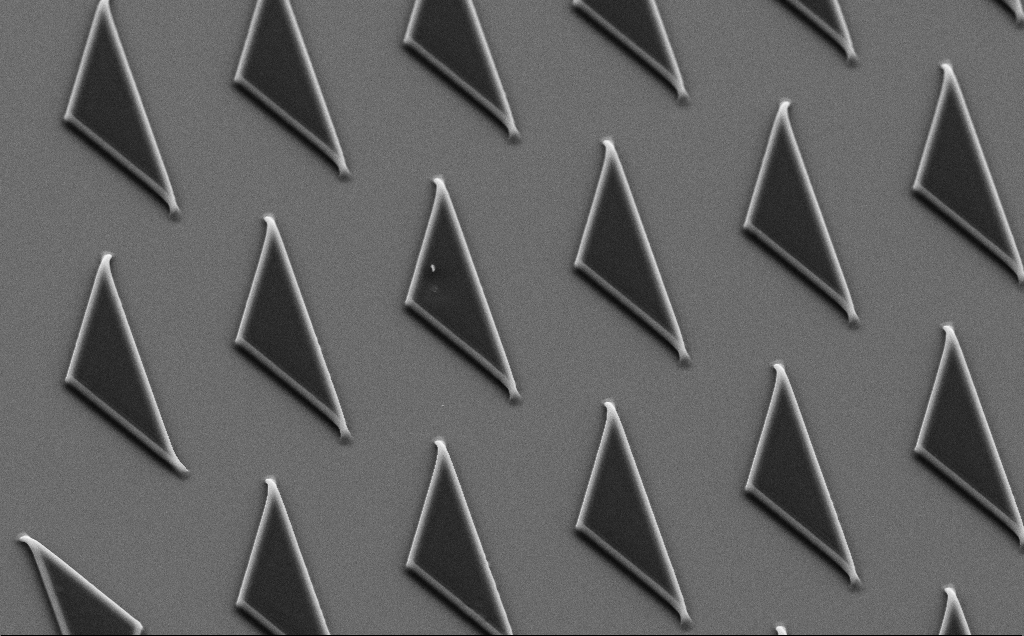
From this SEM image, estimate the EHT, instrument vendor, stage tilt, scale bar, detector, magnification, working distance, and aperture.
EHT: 10 kV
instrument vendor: Zeiss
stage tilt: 35°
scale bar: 10000 nm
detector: SE2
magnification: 2.71 K X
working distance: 8 mm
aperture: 30 µm